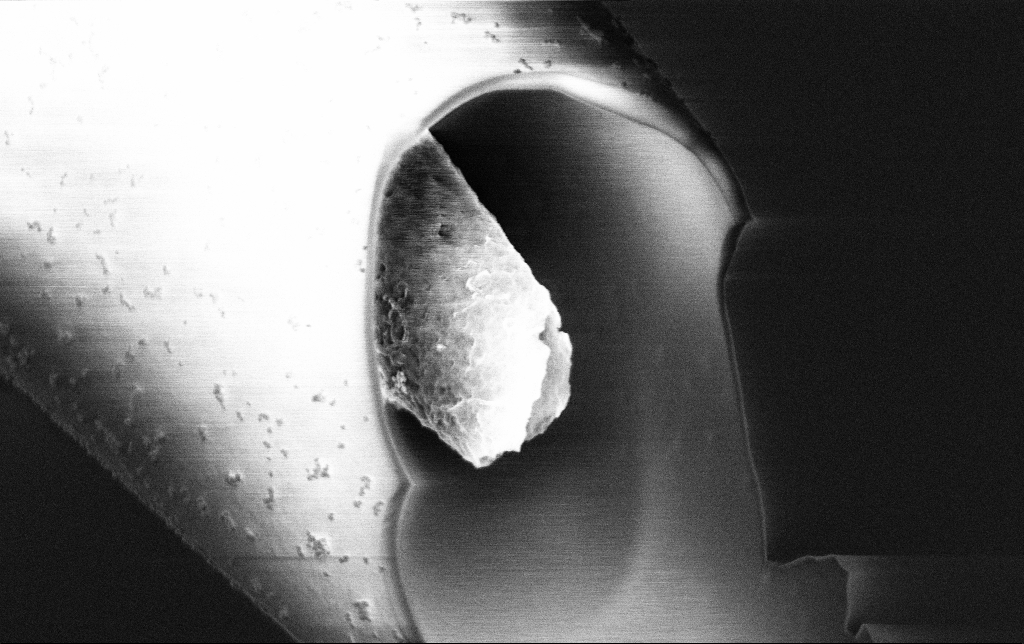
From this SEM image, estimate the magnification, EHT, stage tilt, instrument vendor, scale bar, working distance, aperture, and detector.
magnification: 50 K X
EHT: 3 kV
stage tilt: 45°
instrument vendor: Zeiss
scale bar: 1000 nm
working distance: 7.7 mm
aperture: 30 µm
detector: InLens